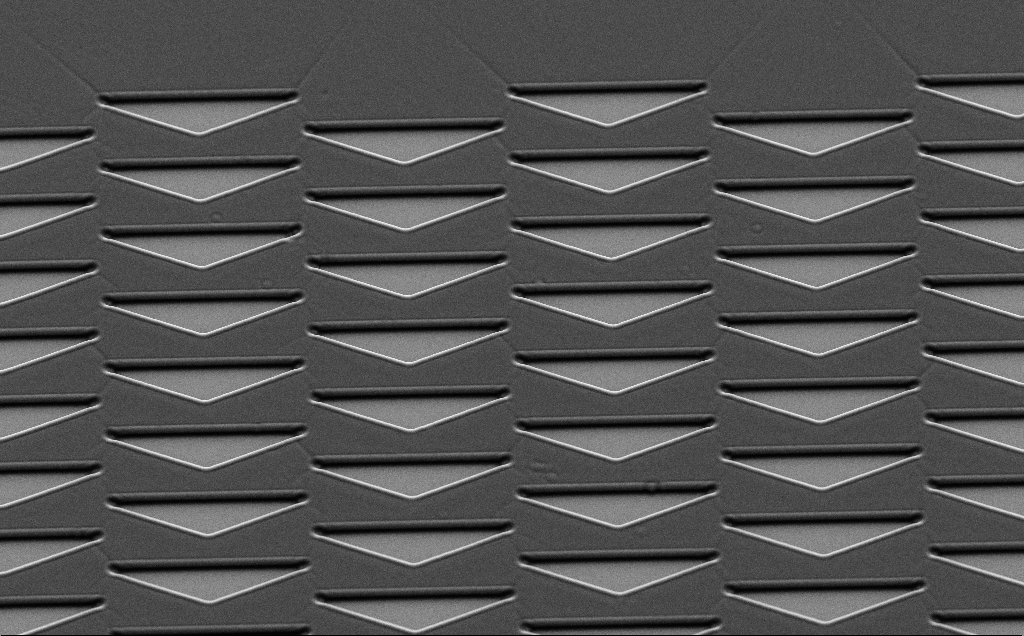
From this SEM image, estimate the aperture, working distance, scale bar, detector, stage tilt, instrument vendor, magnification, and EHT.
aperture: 30 µm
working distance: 6 mm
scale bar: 10000 nm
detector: SE2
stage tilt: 35°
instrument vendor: Zeiss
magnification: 1.78 K X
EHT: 7 kV